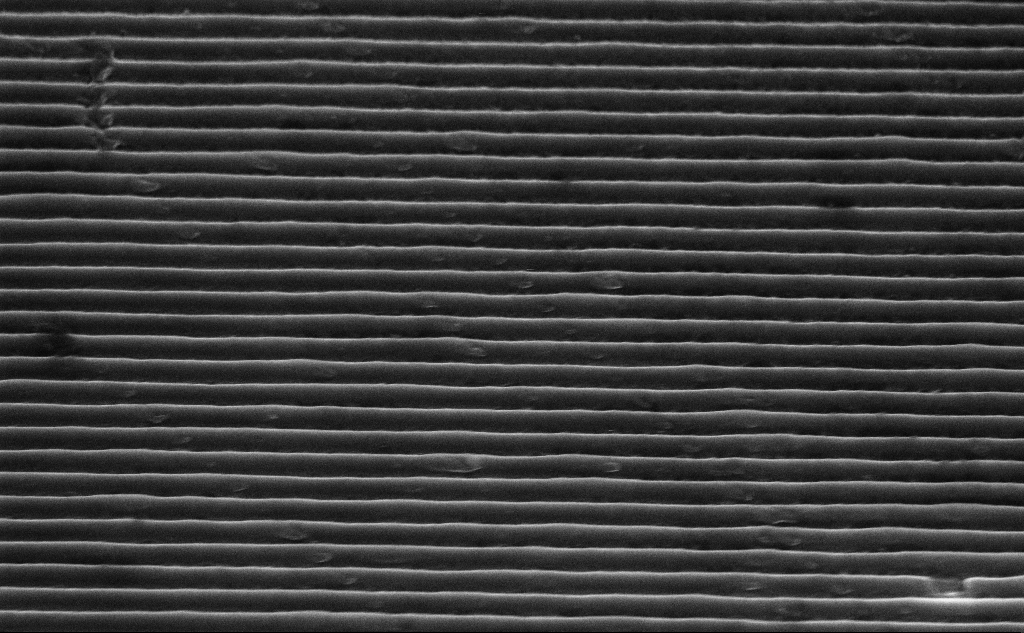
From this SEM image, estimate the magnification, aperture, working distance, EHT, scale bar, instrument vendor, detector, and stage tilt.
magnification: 40.79 K X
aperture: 30 µm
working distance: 7.4 mm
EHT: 5 kV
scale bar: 1000 nm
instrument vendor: Zeiss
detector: InLens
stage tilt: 45°